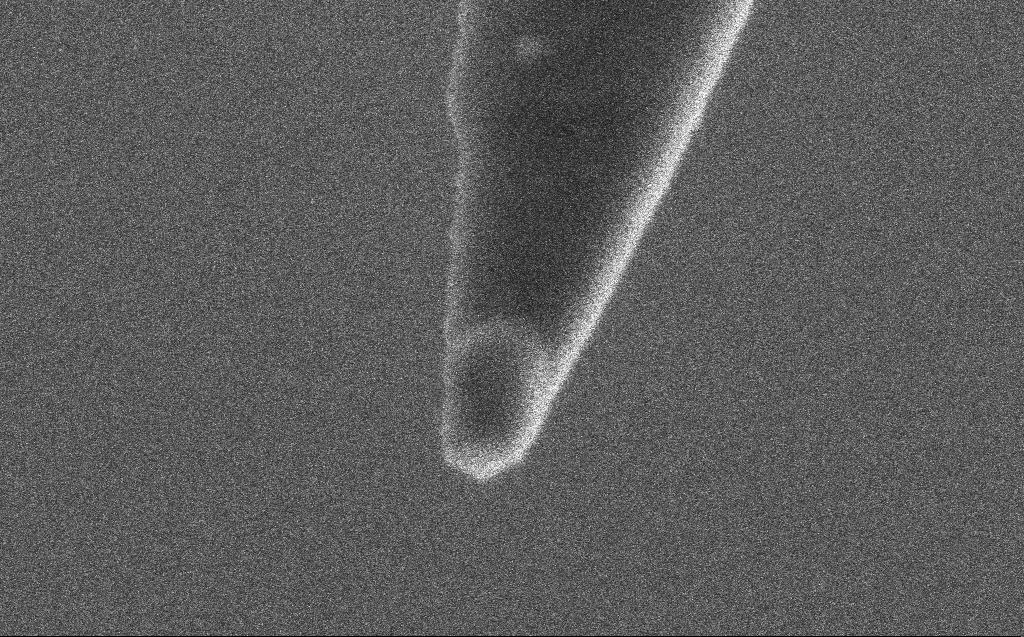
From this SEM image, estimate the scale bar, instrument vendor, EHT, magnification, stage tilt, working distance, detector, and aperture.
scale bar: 200 nm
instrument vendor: Zeiss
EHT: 2 kV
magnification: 250 K X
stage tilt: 45°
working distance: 5 mm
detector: SE2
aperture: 30 µm